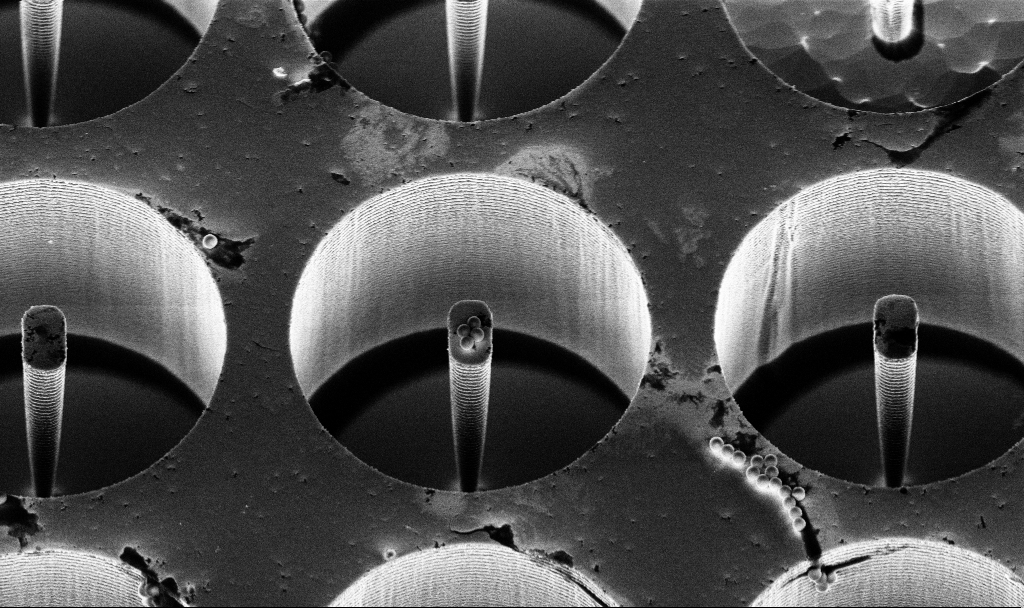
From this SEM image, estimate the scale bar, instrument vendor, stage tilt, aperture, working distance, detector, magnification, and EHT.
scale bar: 10000 nm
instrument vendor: Zeiss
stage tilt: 30°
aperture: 30 µm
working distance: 7.1 mm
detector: InLens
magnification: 6.79 K X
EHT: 5 kV